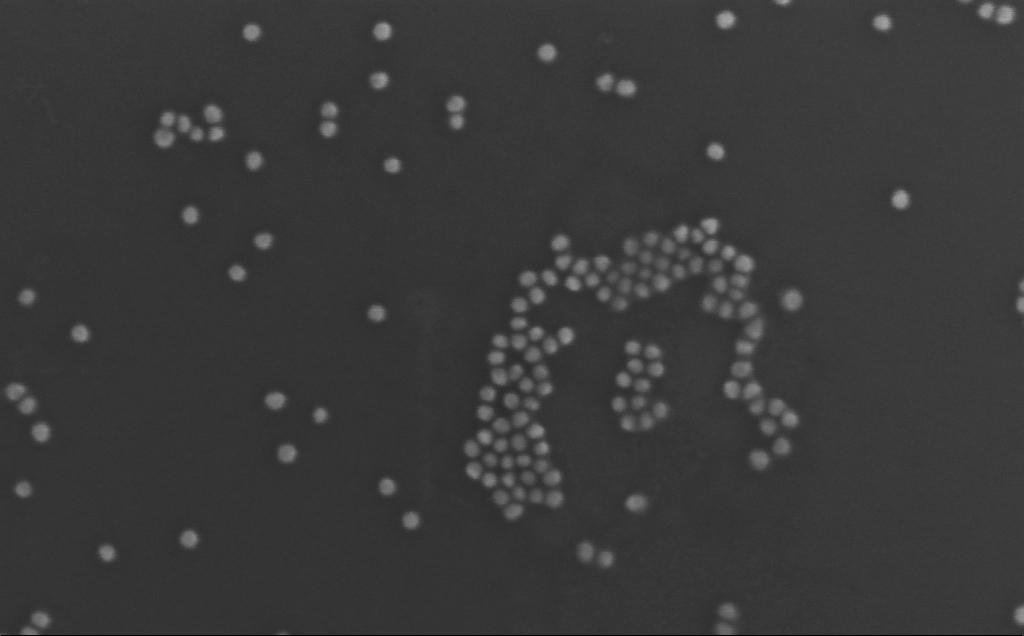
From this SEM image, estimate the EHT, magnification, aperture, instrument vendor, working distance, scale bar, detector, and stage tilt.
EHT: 10 kV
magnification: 371.33 K X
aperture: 30 µm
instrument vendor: Zeiss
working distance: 3 mm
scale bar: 100 nm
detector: InLens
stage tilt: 0°